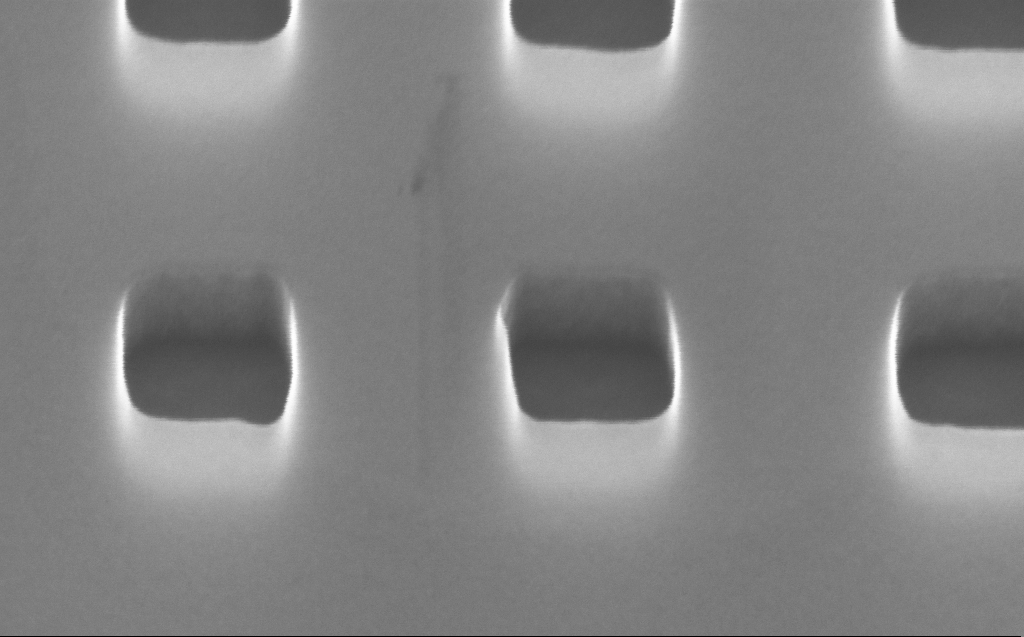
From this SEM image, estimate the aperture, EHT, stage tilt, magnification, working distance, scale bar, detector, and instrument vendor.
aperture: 30 µm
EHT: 10 kV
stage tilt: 45°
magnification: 285.8 K X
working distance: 3 mm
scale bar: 200 nm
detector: InLens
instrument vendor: Zeiss